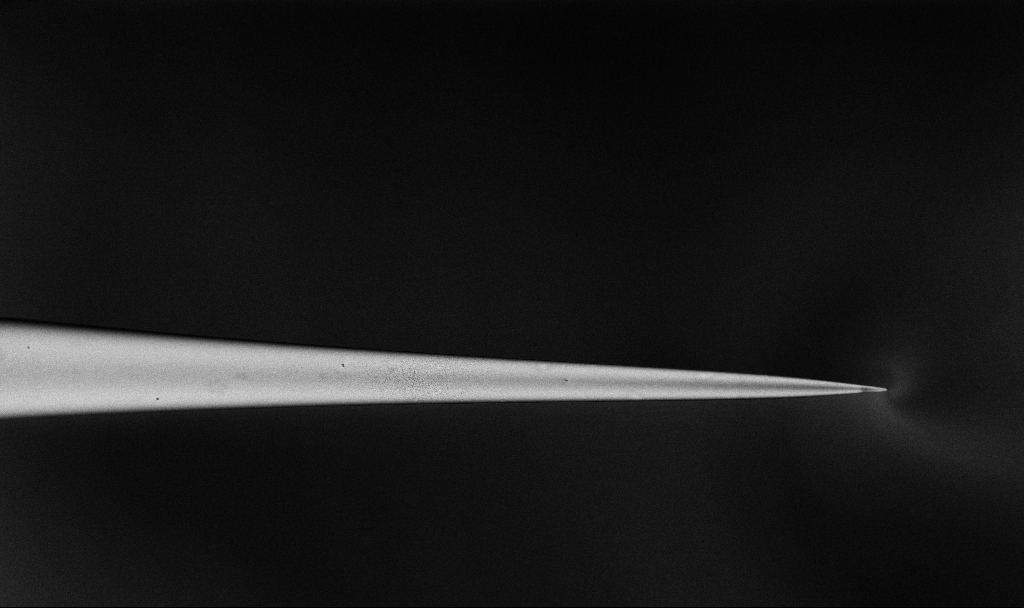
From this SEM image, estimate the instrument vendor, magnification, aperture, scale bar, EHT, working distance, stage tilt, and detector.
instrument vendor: Zeiss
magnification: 1.34 K X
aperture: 30 µm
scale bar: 10000 nm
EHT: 1.5 kV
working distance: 3.3 mm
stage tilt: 45°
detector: InLens